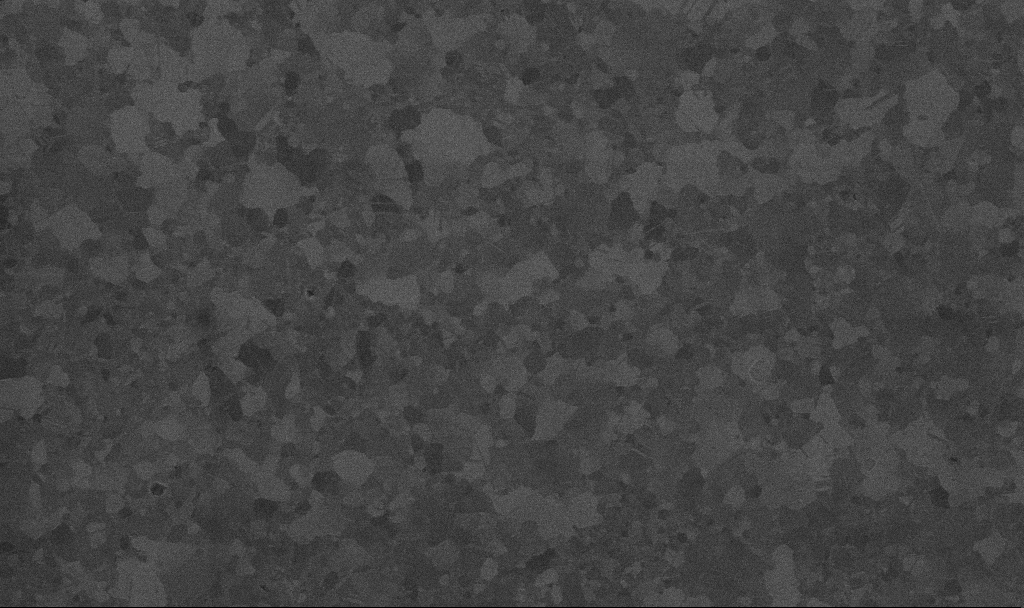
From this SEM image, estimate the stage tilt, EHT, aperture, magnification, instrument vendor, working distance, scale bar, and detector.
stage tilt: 0°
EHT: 10 kV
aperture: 30 µm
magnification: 50 K X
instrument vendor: Zeiss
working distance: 3.4 mm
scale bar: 1000 nm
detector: InLens